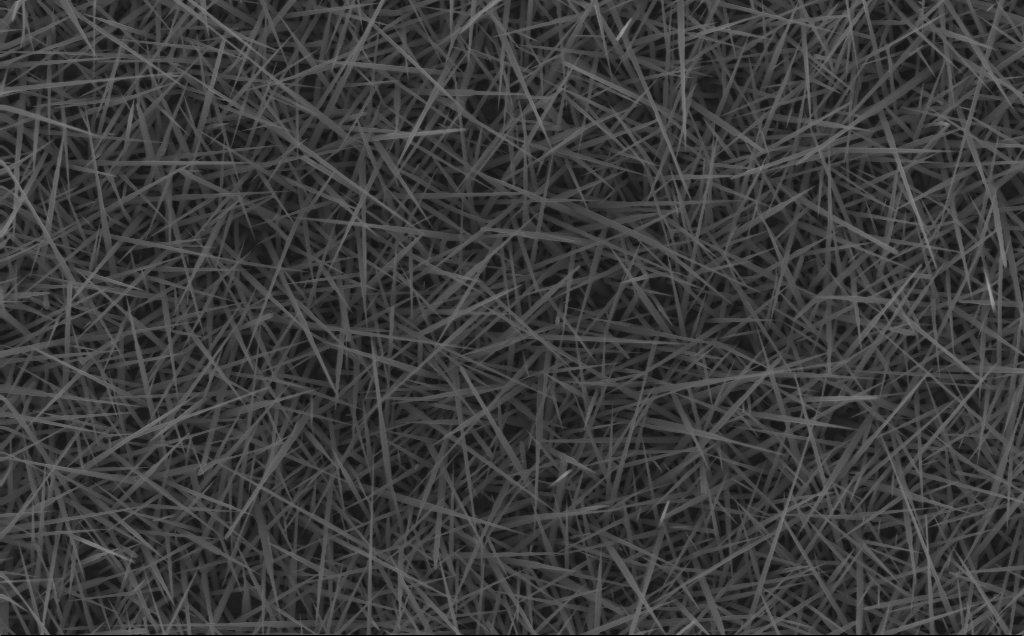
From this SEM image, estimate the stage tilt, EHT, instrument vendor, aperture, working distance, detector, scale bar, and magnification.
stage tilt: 0°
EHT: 10 kV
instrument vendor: Zeiss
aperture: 30 µm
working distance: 4 mm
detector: InLens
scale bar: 2000 nm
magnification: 20 K X